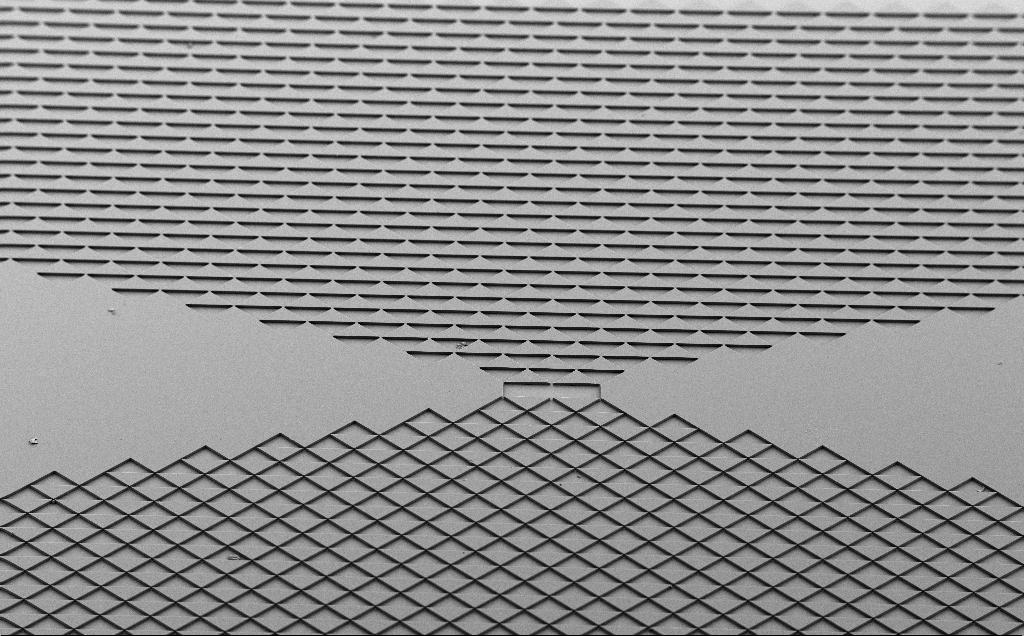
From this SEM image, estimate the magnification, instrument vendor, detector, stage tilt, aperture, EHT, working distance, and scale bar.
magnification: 0.193 K X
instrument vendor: Zeiss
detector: SE2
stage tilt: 40°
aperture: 30 µm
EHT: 5 kV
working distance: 8 mm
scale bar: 100000 nm